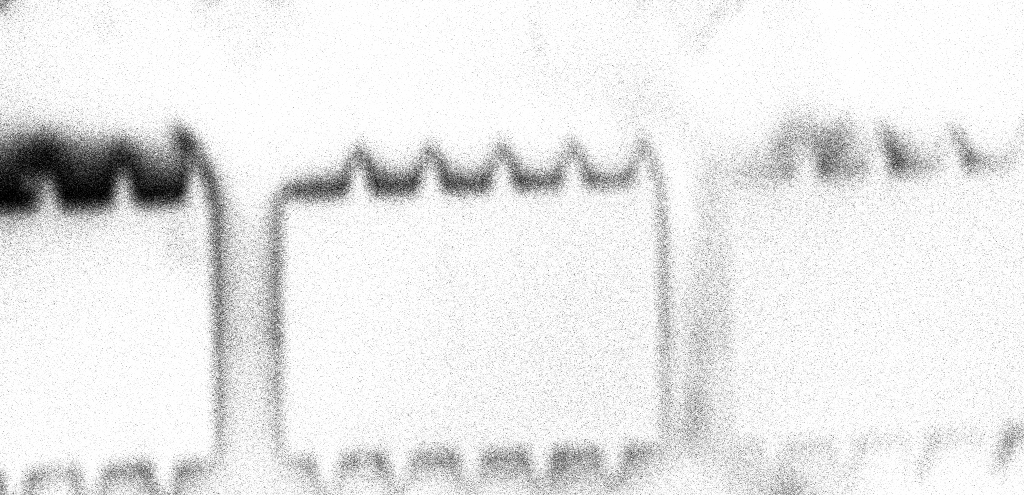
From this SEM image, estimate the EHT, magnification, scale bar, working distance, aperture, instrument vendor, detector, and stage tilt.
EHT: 5 kV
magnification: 0.179 K X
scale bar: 200000 nm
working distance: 4 mm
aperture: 30 µm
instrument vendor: Zeiss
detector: InLens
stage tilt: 0°